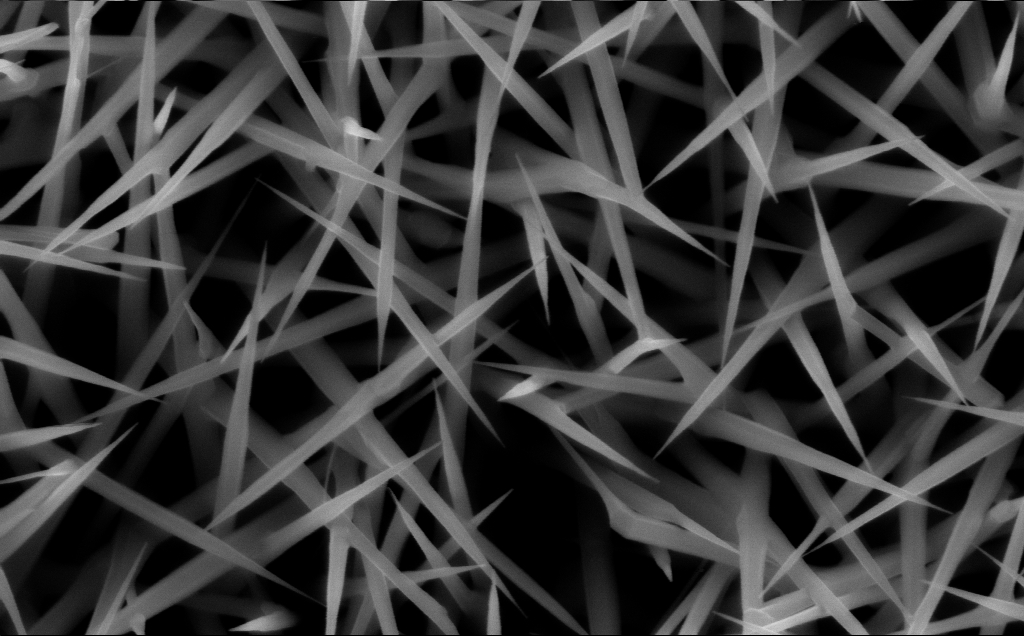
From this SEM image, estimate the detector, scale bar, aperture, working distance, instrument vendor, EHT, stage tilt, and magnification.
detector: InLens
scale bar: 200 nm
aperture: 30 µm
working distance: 7 mm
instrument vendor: Zeiss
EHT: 10 kV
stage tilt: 0°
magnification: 80 K X